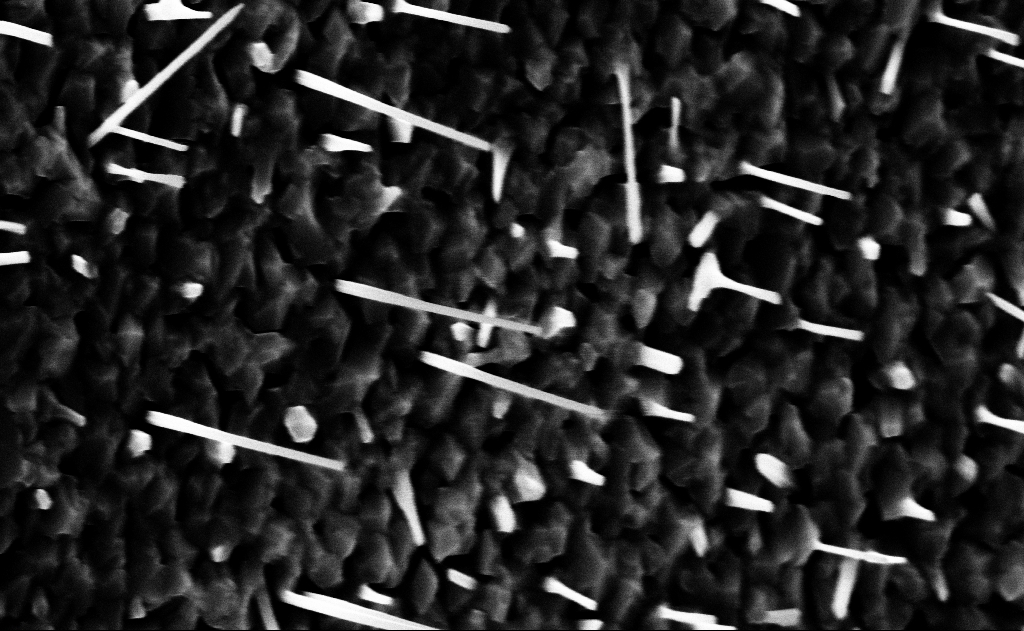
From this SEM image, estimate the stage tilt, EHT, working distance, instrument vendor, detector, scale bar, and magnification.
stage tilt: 0°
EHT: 10 kV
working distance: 14 mm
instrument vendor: Zeiss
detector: InLens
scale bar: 1000 nm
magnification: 40 K X